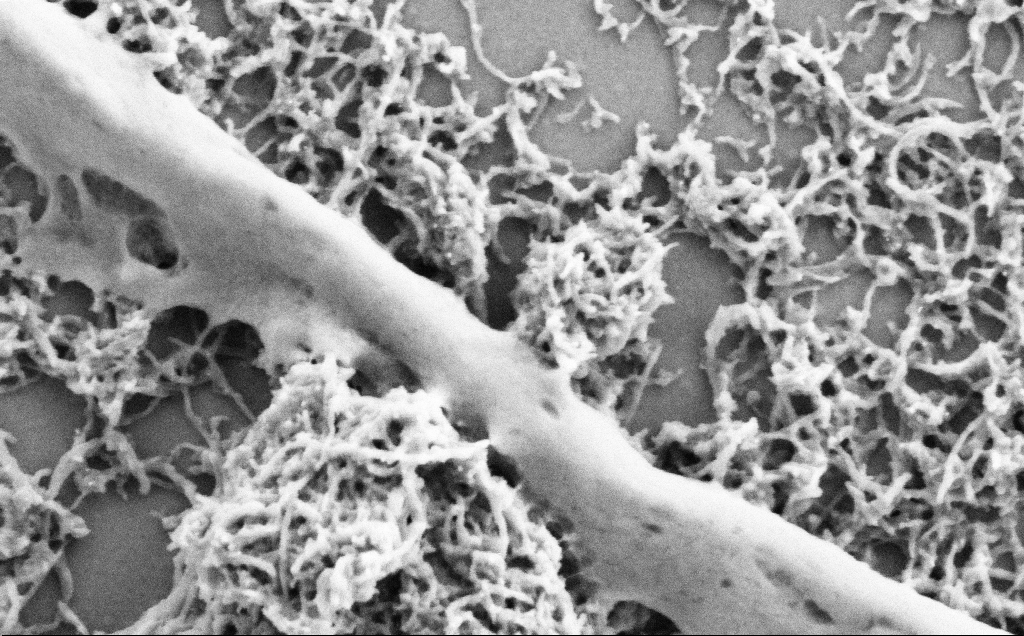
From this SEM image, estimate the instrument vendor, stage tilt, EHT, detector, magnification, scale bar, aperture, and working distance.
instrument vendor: Zeiss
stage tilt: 0°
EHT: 2 kV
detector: SE2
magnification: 75 K X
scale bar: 200 nm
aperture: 30 µm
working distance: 7.1 mm